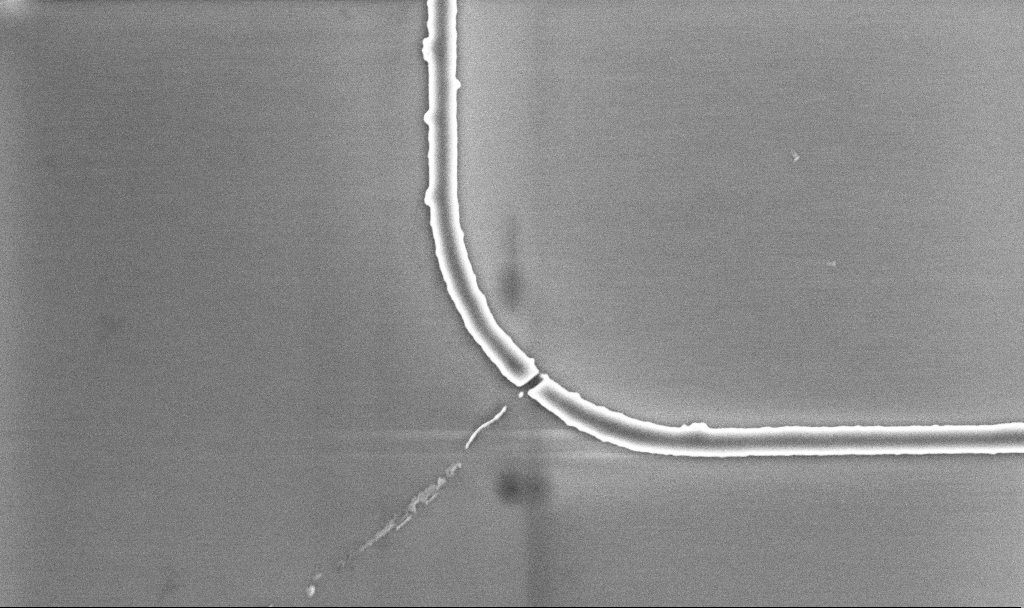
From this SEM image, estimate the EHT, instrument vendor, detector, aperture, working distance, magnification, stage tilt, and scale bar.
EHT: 5 kV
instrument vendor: Zeiss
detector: InLens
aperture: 30 µm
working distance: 5.2 mm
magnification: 19.76 K X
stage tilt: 0°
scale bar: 2000 nm